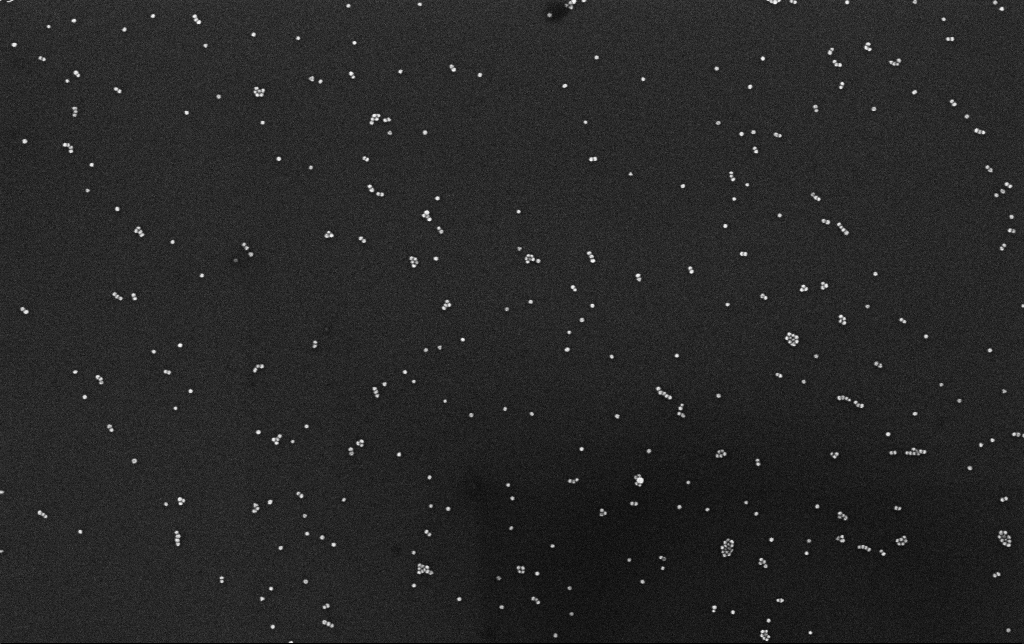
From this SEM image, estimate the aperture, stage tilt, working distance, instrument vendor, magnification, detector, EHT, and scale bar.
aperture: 30 µm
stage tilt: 0°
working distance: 3.1 mm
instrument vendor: Zeiss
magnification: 100 K X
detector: InLens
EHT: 10 kV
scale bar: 200 nm